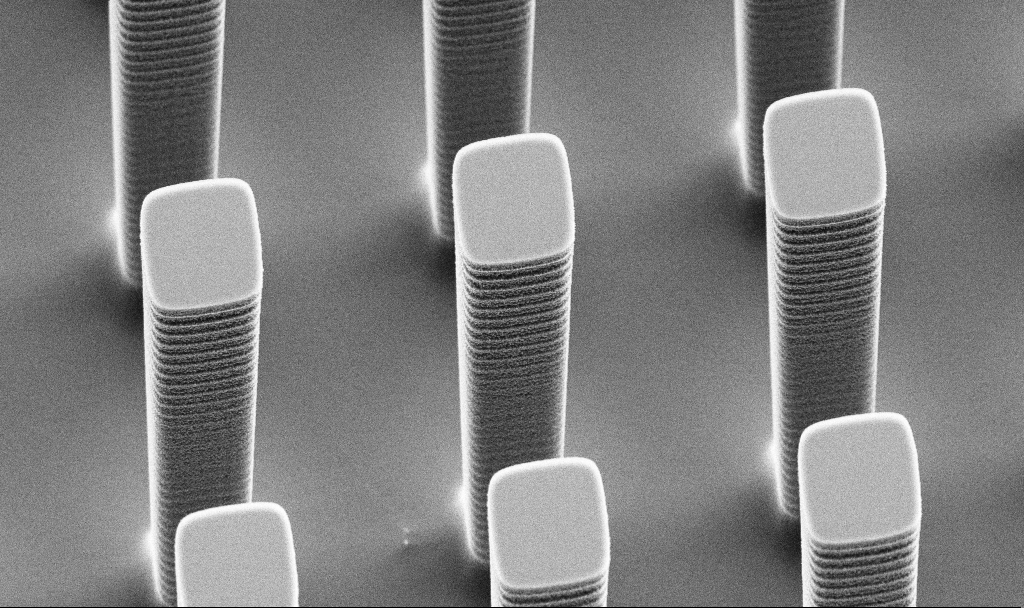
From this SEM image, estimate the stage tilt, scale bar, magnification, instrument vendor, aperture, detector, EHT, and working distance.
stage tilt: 45°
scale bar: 2000 nm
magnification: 9.6 K X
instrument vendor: Zeiss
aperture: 30 µm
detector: SE2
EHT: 5 kV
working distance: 9.7 mm